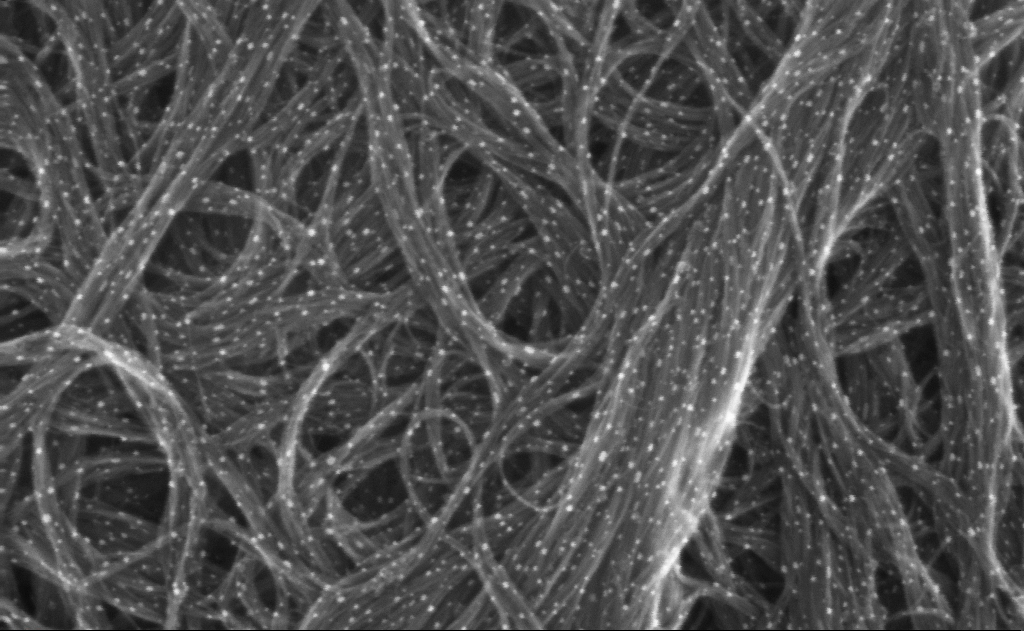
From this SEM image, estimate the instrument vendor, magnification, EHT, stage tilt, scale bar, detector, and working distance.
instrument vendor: Zeiss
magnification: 407.04 K X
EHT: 10 kV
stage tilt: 0°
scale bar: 100 nm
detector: InLens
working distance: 3 mm